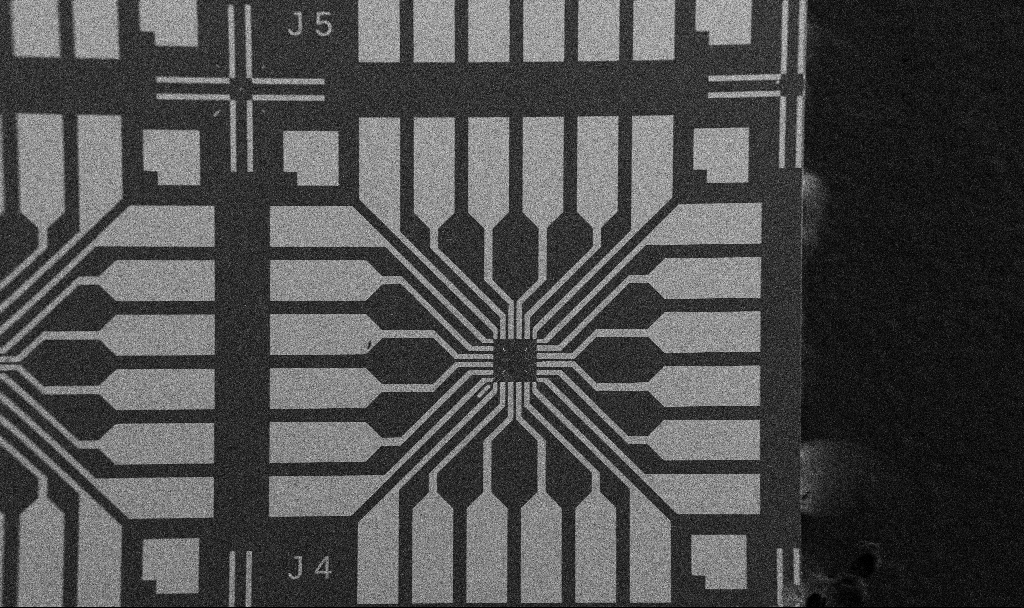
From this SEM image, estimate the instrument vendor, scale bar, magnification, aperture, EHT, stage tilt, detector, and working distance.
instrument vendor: Zeiss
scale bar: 200000 nm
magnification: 0.1 K X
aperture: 30 µm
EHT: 5 kV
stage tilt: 0°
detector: SE2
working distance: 10.7 mm